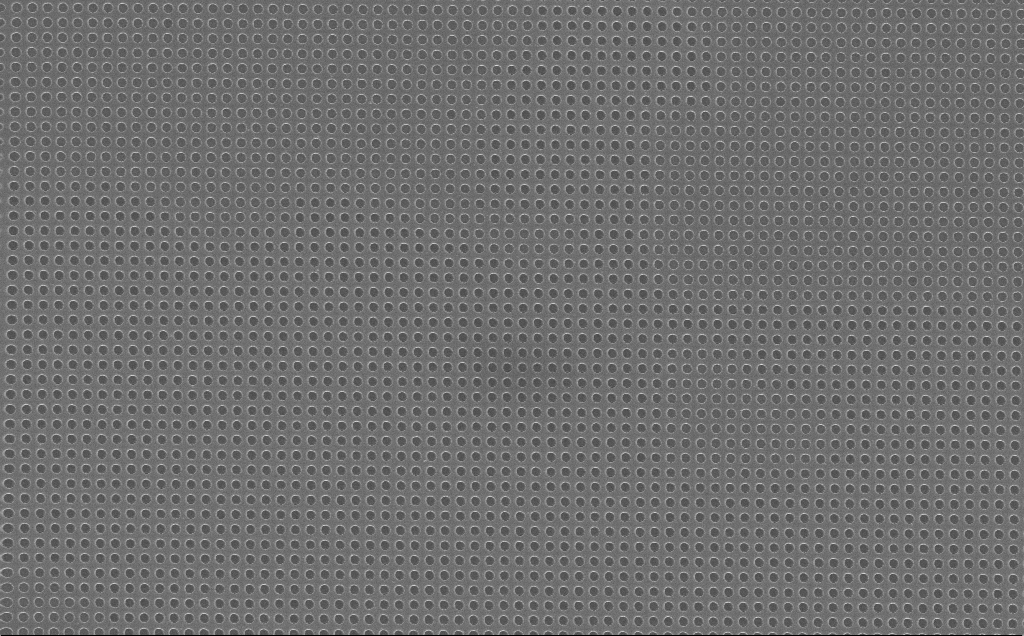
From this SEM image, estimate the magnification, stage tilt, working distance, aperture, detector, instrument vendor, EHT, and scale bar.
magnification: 15.74 K X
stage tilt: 0°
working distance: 7 mm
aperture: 30 µm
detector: InLens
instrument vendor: Zeiss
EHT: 10 kV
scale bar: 2000 nm